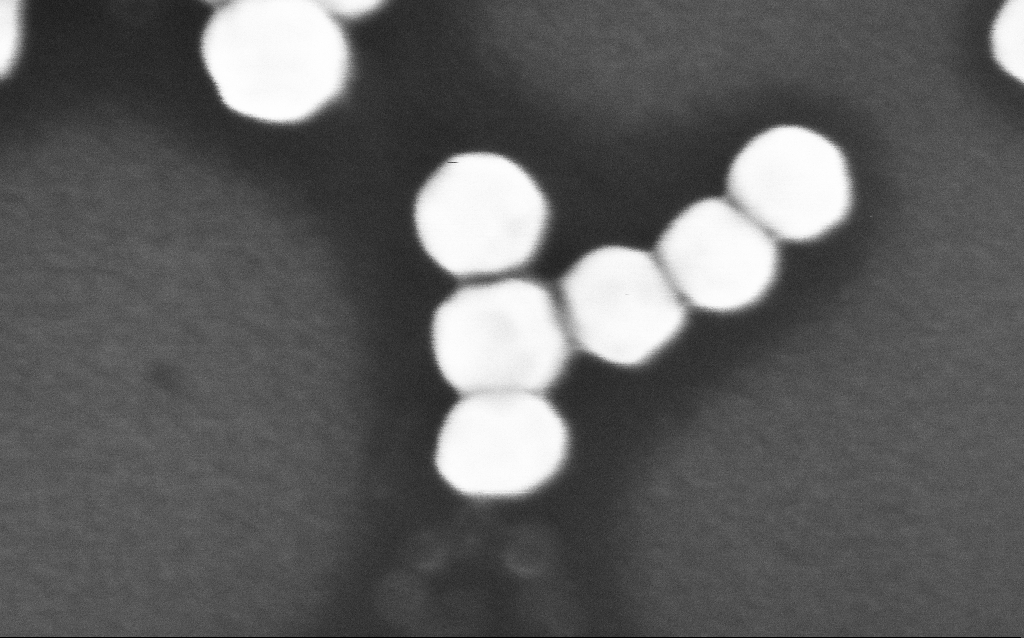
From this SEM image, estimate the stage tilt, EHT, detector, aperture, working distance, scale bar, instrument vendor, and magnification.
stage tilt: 0°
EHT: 8 kV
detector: InLens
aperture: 30 µm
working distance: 3.1 mm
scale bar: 100 nm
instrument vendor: Zeiss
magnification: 690.93 K X